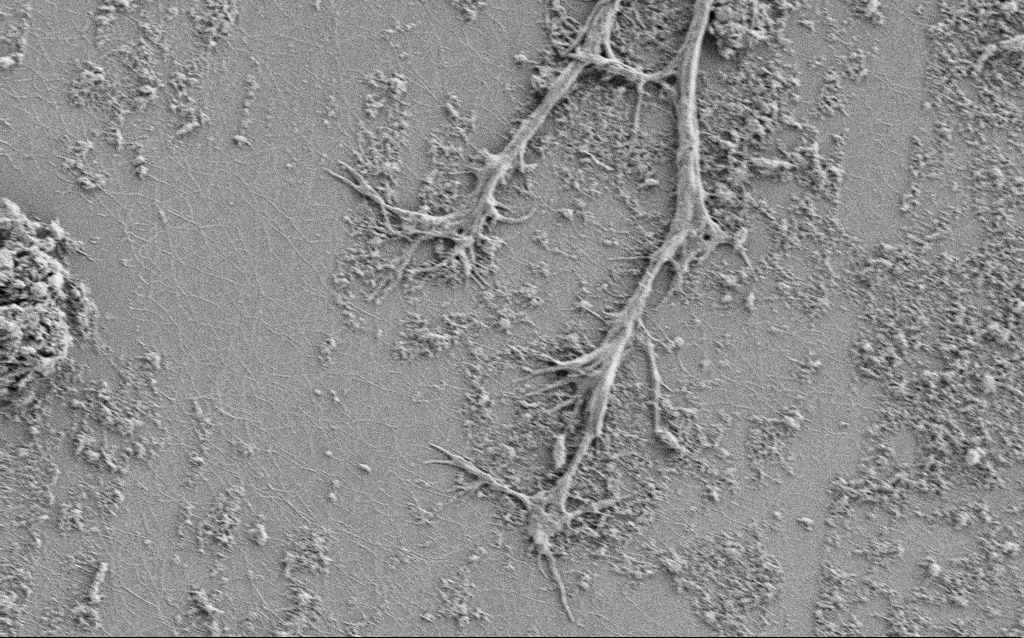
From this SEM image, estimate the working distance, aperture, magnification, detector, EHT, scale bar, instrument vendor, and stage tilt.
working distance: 4 mm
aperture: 30 µm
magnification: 5 K X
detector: SE2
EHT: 1 kV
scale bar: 10000 nm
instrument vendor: Zeiss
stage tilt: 0°